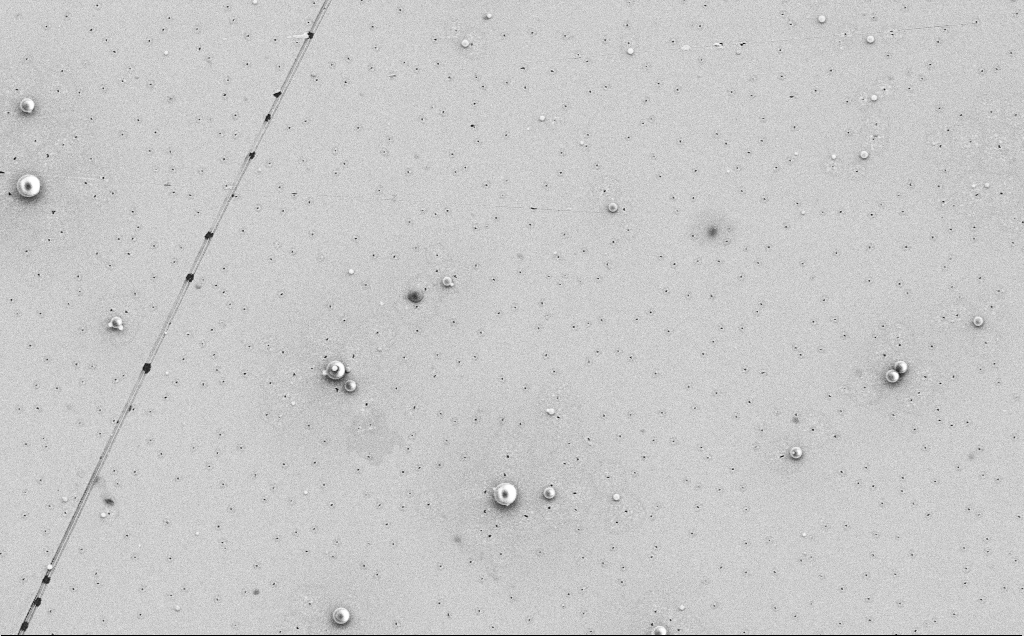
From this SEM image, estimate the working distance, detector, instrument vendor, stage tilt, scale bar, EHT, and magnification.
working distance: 12 mm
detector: SE2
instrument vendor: Zeiss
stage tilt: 0°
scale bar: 10000 nm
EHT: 5 kV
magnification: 4.2 K X